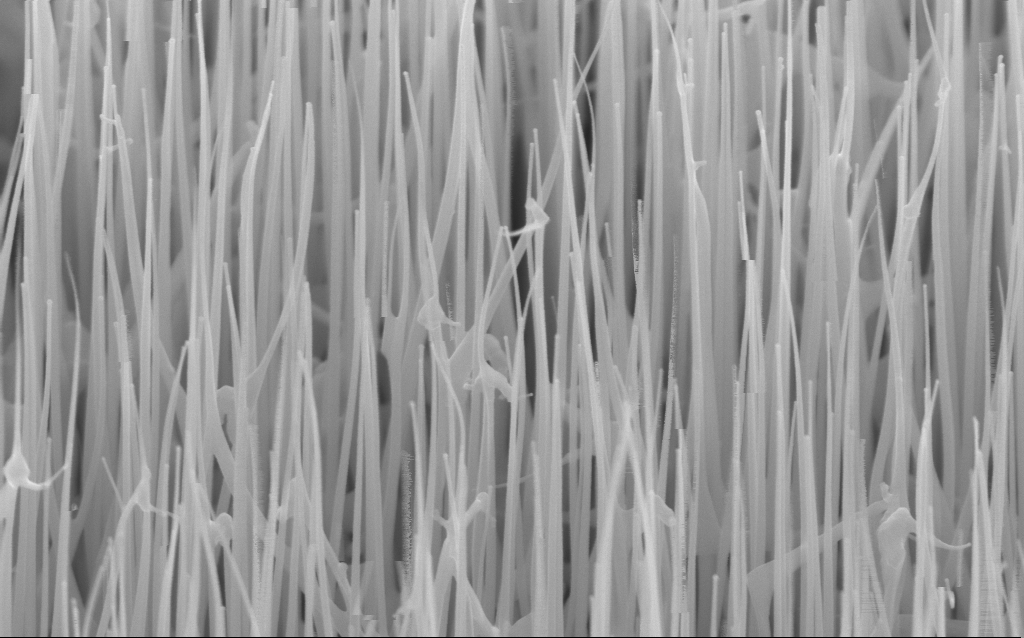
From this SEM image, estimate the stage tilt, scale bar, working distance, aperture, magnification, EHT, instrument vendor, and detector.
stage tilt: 45°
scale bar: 200 nm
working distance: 6 mm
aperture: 30 µm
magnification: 80 K X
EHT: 10 kV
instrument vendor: Zeiss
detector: InLens